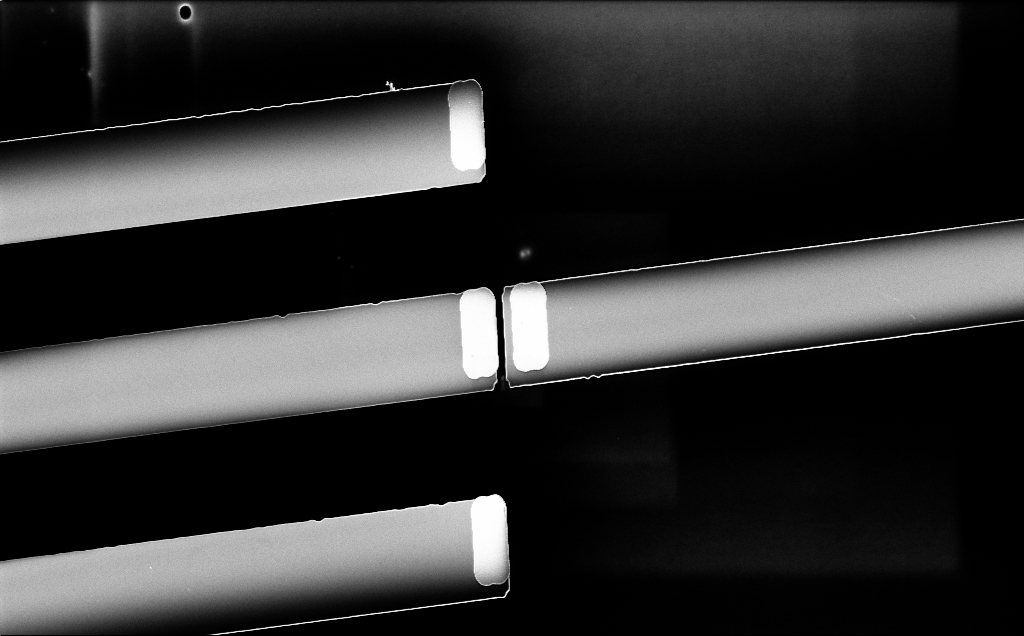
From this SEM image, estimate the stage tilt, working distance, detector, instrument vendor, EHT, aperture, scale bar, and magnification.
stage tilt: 0°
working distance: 6 mm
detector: InLens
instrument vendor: Zeiss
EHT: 5 kV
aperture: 30 µm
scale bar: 10000 nm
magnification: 1.39 K X